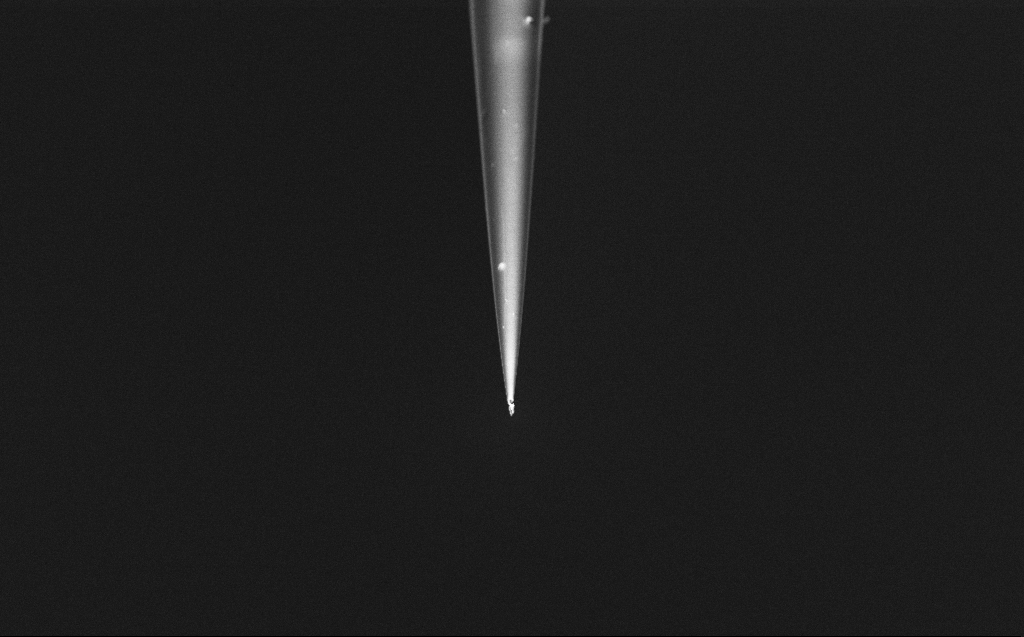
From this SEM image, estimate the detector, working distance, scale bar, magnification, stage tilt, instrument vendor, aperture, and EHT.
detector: InLens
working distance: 6 mm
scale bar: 2000 nm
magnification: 10 K X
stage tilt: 45°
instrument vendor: Zeiss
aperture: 30 µm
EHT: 2 kV